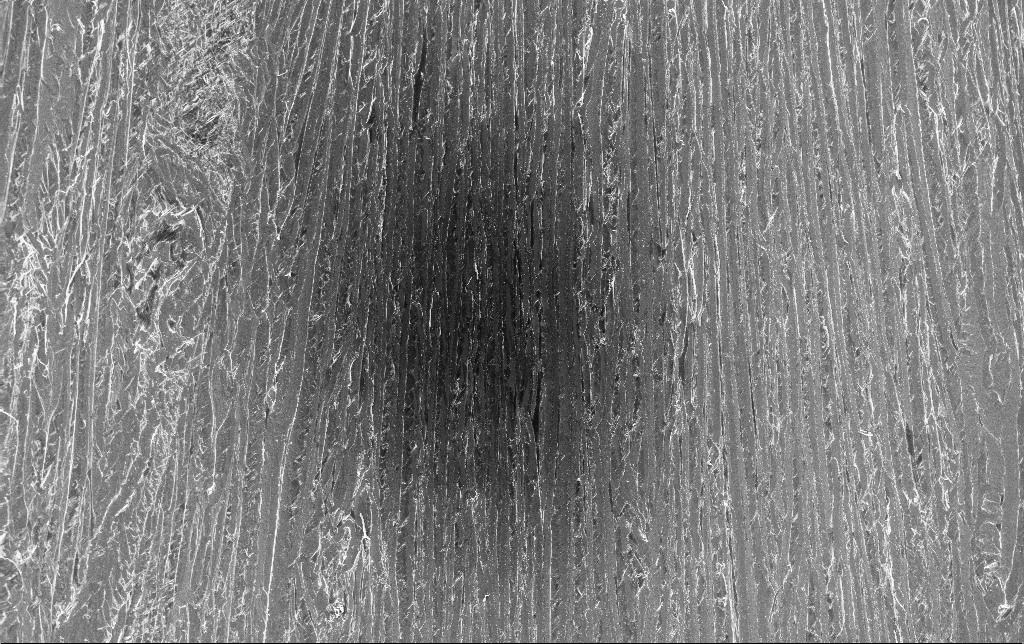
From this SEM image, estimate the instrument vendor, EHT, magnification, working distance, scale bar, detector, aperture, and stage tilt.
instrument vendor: Zeiss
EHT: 10 kV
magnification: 0.213 K X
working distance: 3.4 mm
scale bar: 100000 nm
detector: InLens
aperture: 30 µm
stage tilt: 0°